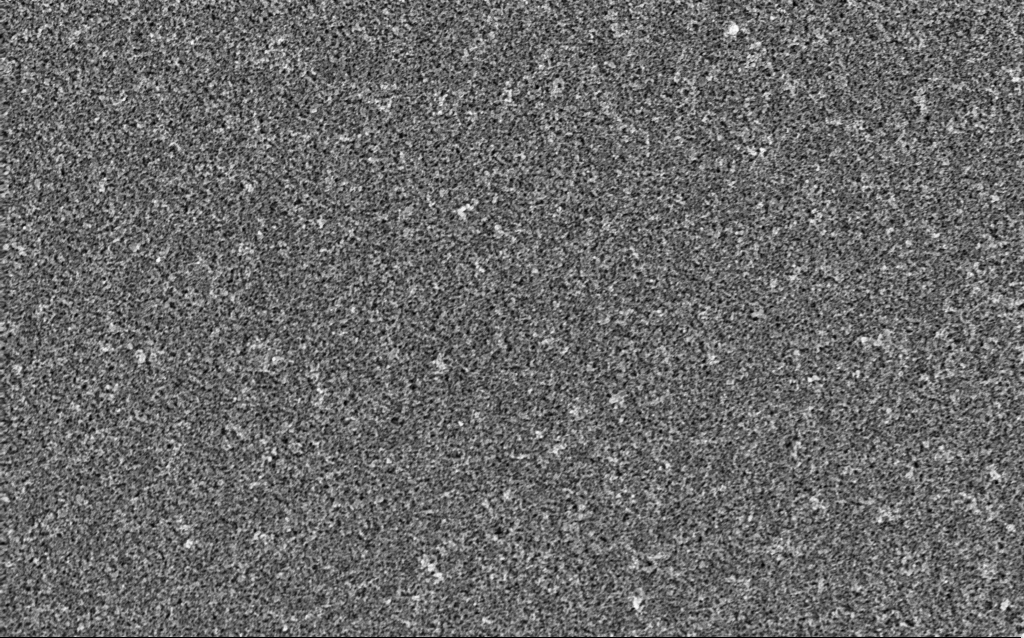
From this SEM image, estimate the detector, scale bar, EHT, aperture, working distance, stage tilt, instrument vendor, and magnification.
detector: InLens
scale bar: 1000 nm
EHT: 5 kV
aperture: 30 µm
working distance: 4.9 mm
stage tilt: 0°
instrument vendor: Zeiss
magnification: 20.87 K X